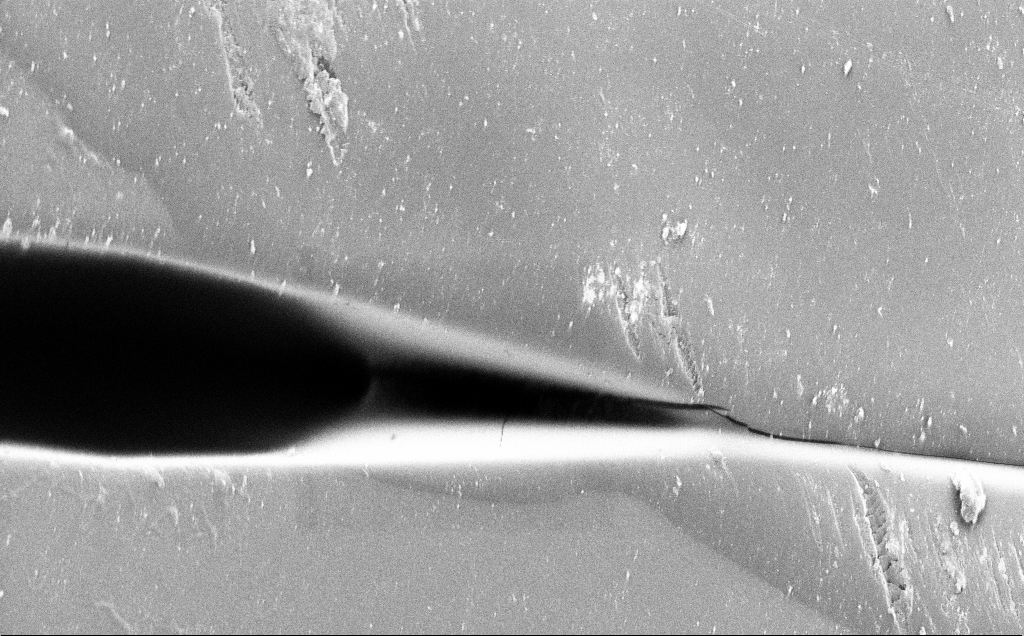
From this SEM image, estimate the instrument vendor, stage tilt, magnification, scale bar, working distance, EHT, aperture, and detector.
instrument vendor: Zeiss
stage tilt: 0°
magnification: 2.39 K X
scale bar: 20000 nm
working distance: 5 mm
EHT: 1.2 kV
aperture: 120 µm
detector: SE2